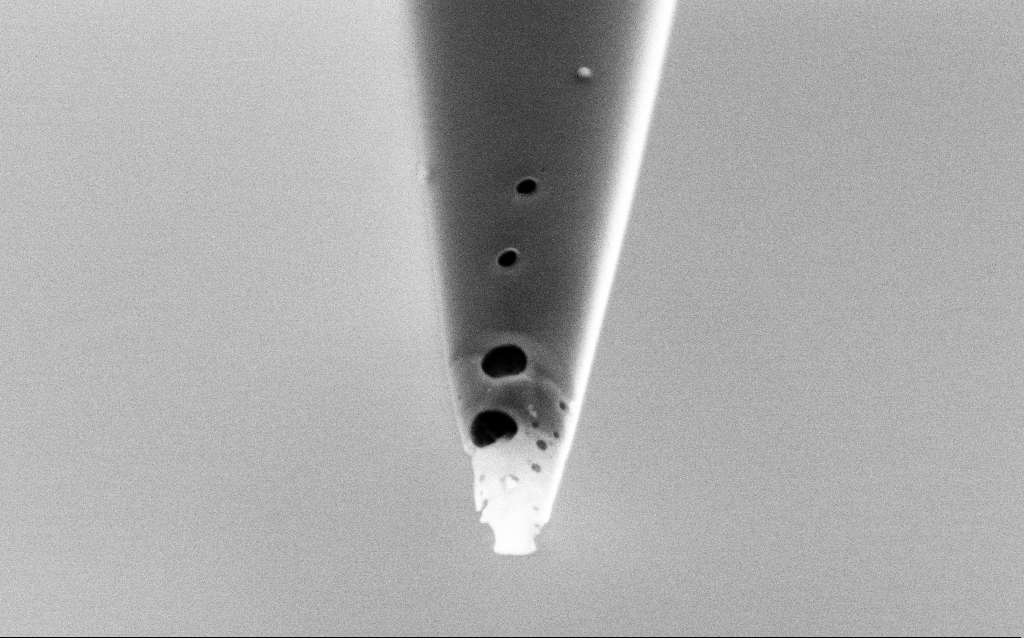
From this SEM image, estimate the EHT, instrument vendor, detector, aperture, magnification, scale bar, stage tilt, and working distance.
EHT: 2 kV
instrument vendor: Zeiss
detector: SE2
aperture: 30 µm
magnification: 75 K X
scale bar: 200 nm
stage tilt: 45°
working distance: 6 mm